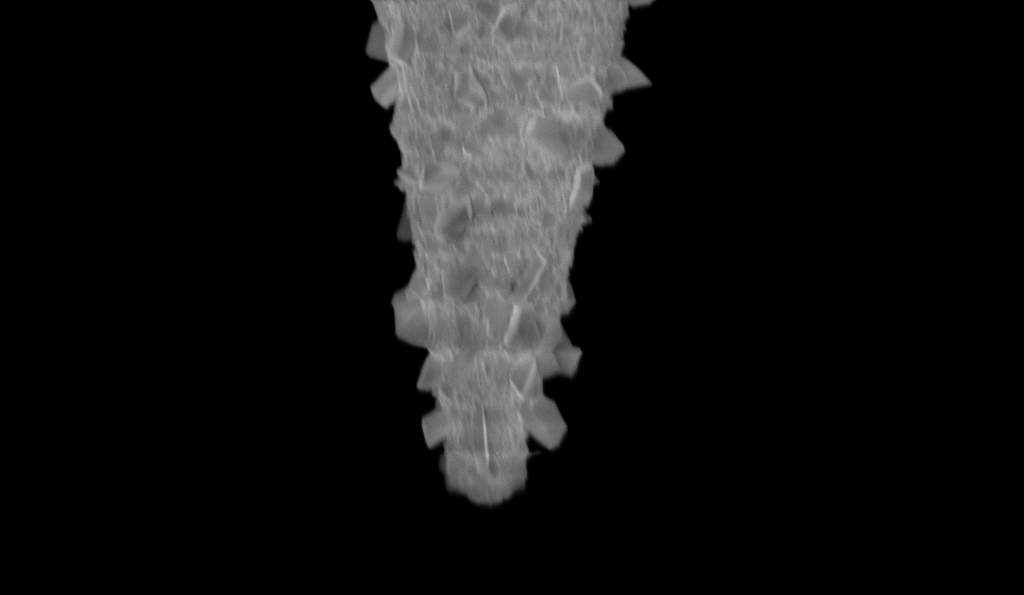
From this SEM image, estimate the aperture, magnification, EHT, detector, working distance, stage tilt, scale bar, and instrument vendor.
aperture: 30 µm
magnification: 25 K X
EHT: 1 kV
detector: InLens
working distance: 6.7 mm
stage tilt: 0°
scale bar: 2000 nm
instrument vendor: Zeiss